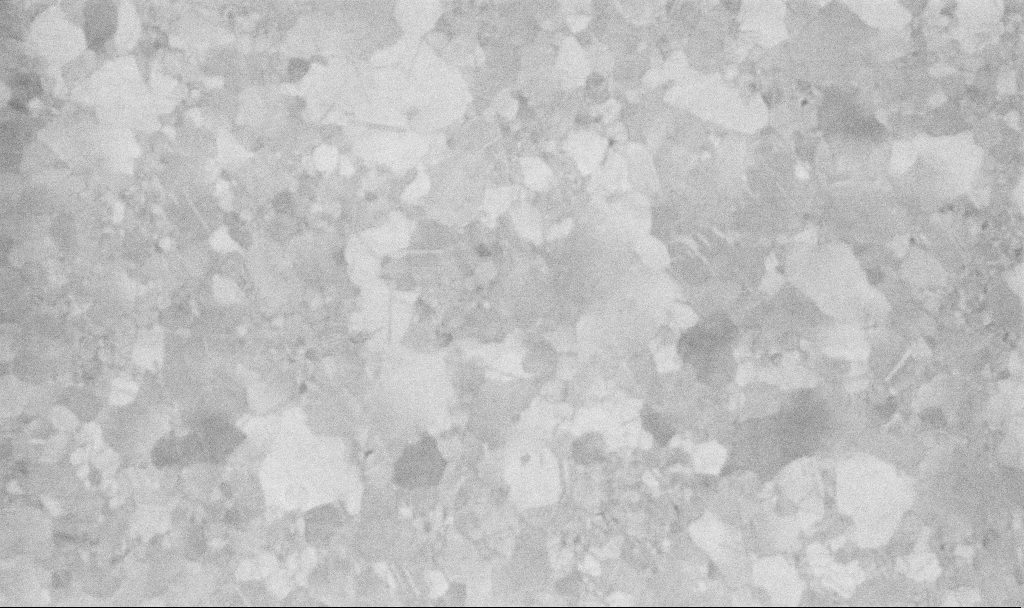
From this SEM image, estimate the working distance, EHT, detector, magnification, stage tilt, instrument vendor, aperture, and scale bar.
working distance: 3.4 mm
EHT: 10 kV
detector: InLens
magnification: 100 K X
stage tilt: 0°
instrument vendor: Zeiss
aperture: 30 µm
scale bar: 200 nm